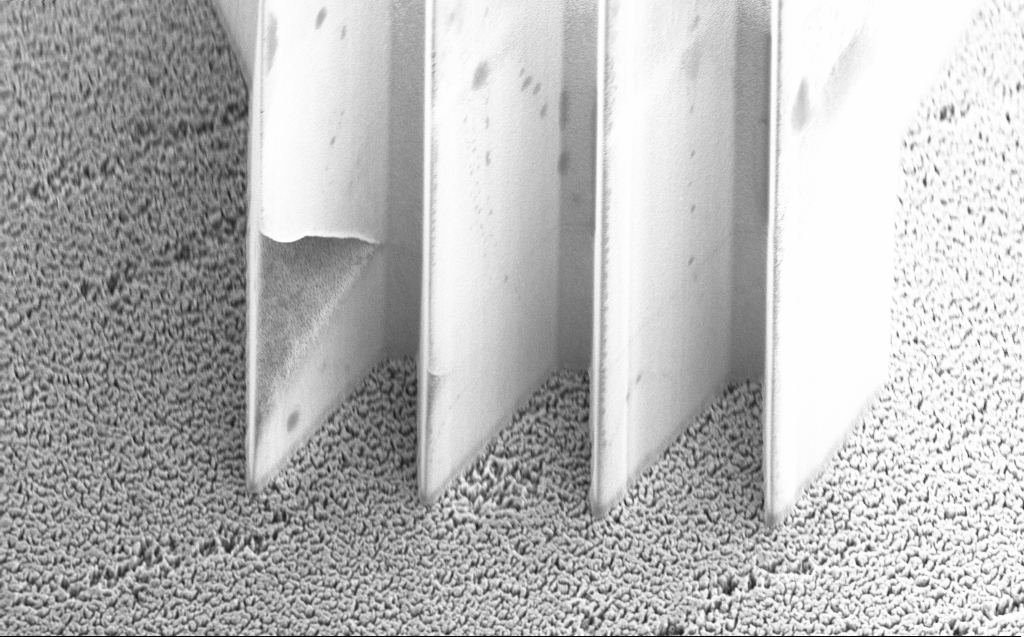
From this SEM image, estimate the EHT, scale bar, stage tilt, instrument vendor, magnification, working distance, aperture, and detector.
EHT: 5 kV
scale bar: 10000 nm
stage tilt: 45°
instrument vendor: Zeiss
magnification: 5.65 K X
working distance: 5 mm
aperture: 30 µm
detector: InLens